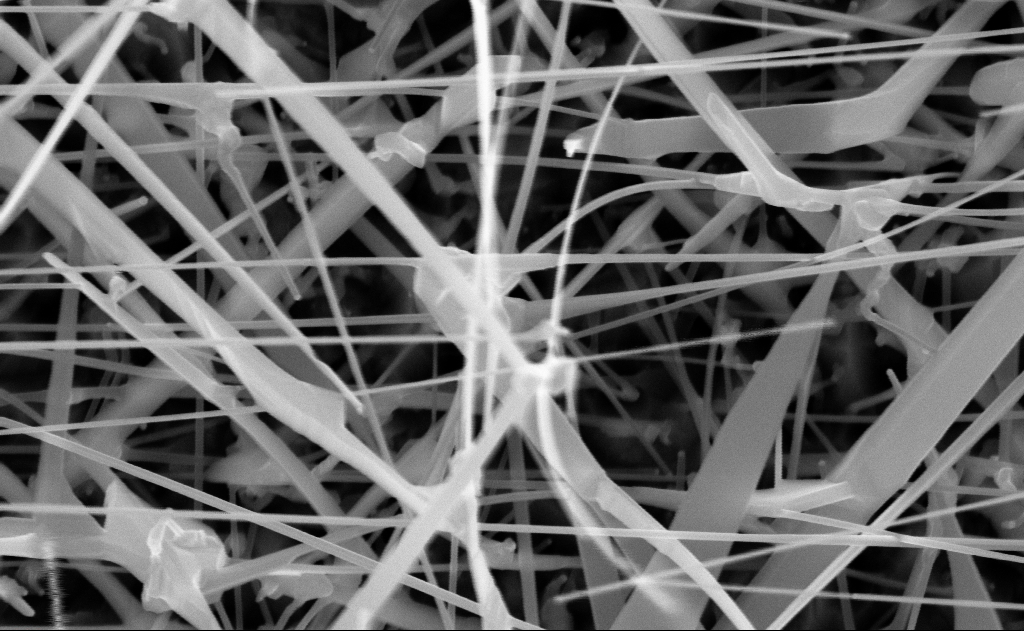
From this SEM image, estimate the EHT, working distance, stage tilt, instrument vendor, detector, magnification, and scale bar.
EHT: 10 kV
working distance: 11 mm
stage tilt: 0°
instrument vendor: Zeiss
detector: InLens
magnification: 80 K X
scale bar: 200 nm